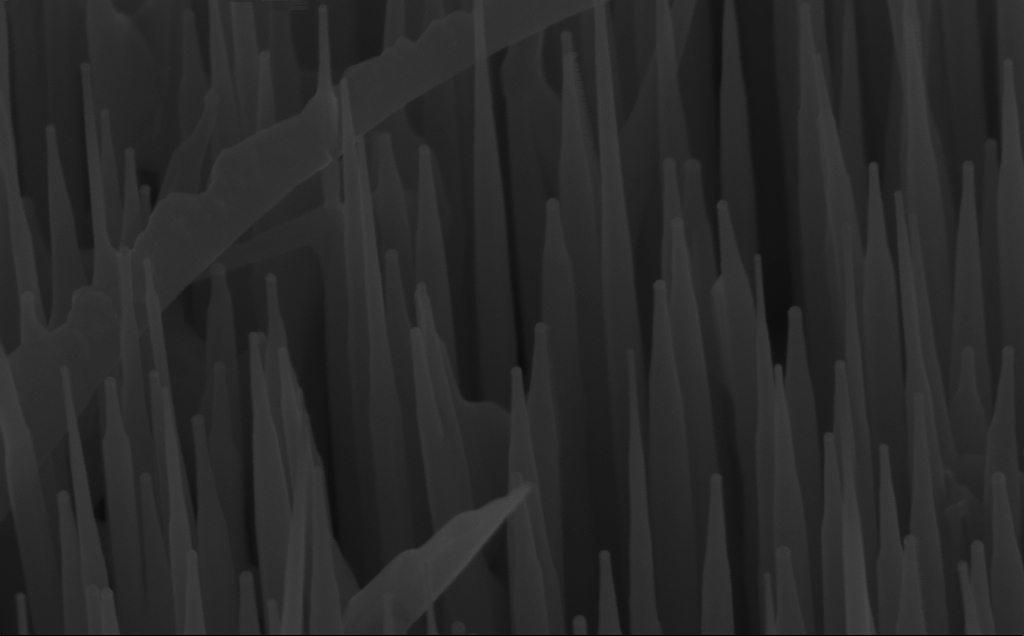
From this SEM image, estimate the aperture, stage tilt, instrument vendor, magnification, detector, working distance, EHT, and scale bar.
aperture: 30 µm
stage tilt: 45°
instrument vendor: Zeiss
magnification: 150 K X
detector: InLens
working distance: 6 mm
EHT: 10 kV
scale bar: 100 nm